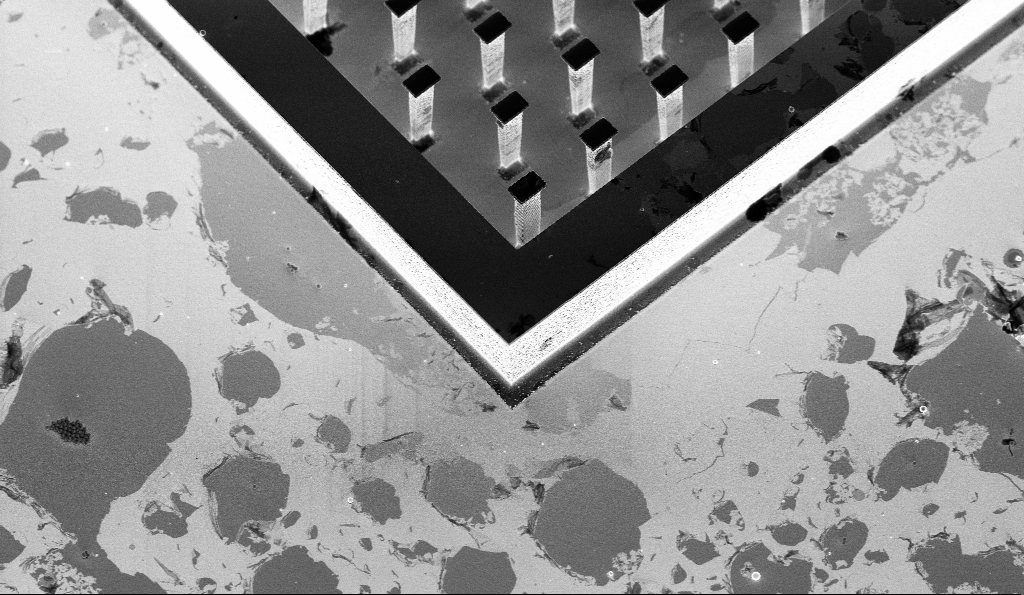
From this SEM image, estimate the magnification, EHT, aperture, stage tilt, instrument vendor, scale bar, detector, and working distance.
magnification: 2.85 K X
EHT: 3 kV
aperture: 30 µm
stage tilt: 30°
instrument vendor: Zeiss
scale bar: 10000 nm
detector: InLens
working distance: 5.5 mm